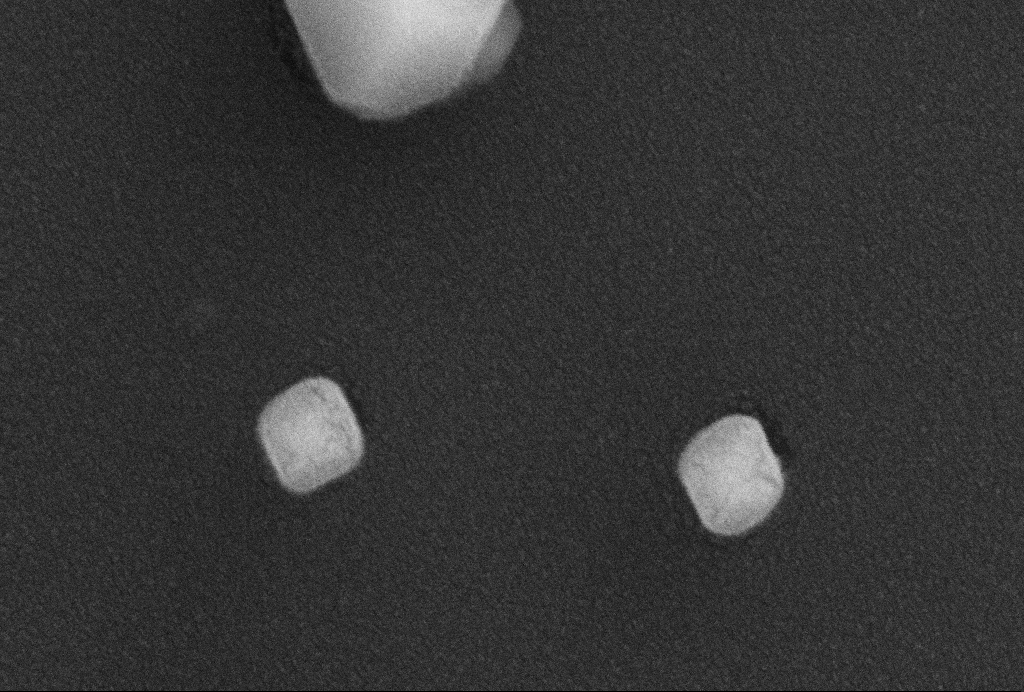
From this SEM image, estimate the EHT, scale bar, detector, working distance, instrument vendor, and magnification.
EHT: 5 kV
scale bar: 200 nm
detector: SE2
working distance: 2.6 mm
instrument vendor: Zeiss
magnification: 250 K X